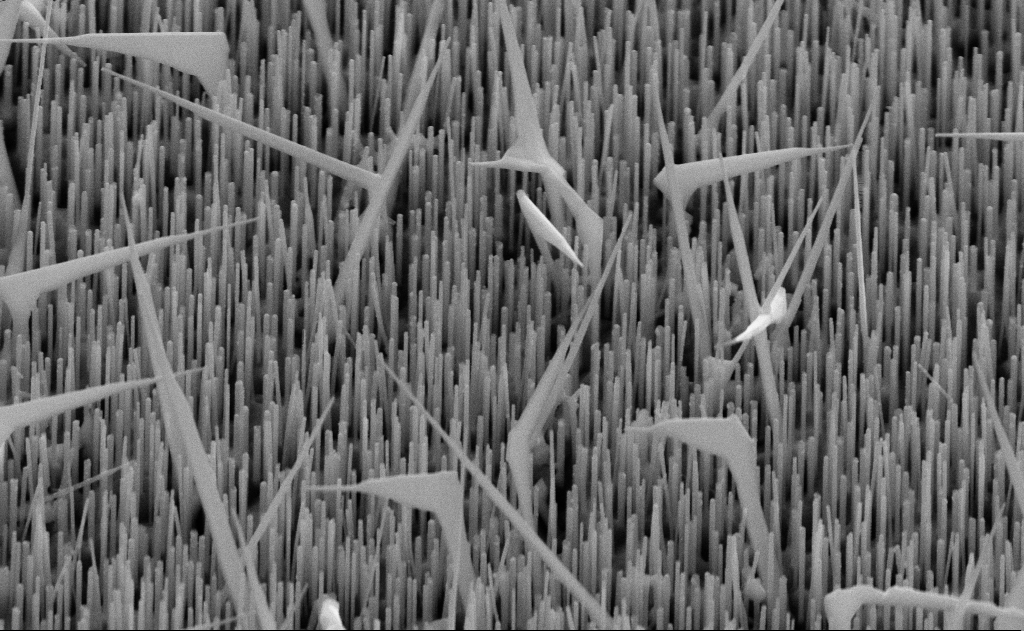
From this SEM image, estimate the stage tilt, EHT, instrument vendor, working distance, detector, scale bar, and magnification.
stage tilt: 45°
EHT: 10 kV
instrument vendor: Zeiss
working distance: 11 mm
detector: SE2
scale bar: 1000 nm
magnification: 40 K X